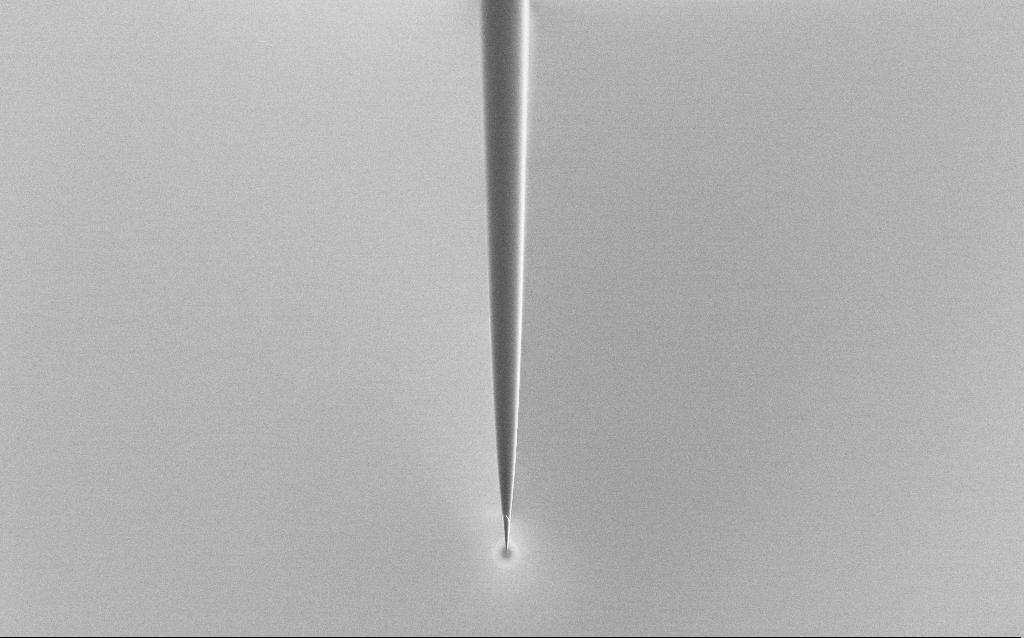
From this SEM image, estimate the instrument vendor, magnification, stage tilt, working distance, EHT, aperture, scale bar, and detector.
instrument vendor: Zeiss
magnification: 0.5 K X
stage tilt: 45°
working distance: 6 mm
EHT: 1 kV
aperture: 30 µm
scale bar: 100000 nm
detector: SE2